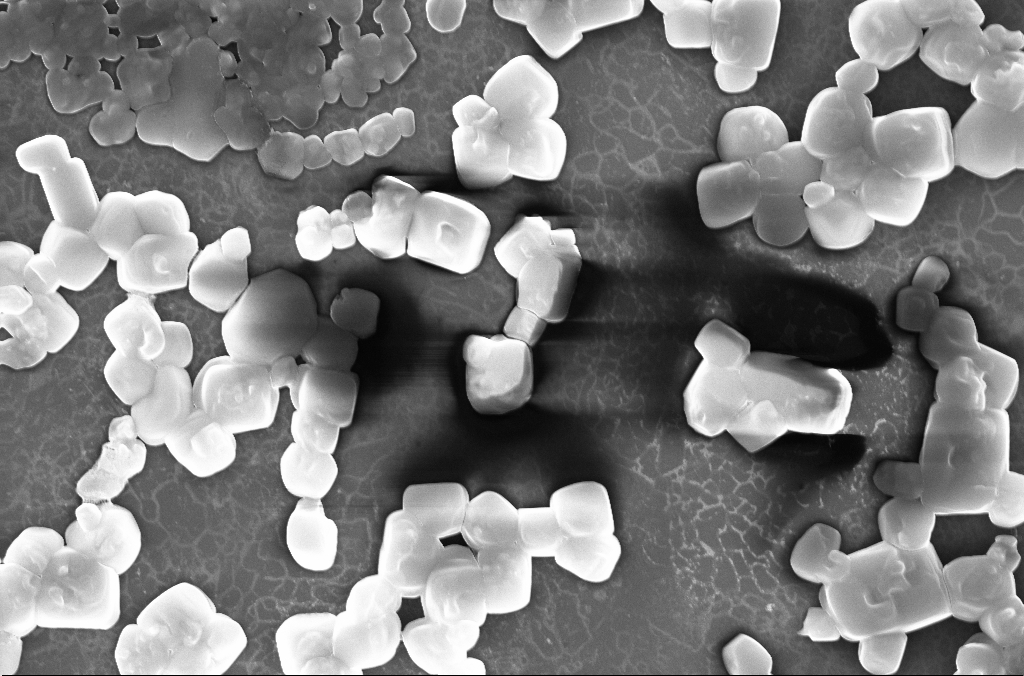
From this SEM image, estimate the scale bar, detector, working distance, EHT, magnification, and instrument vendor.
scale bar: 2000 nm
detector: InLens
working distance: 2.8 mm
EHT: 20 kV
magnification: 10 K X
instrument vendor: Zeiss